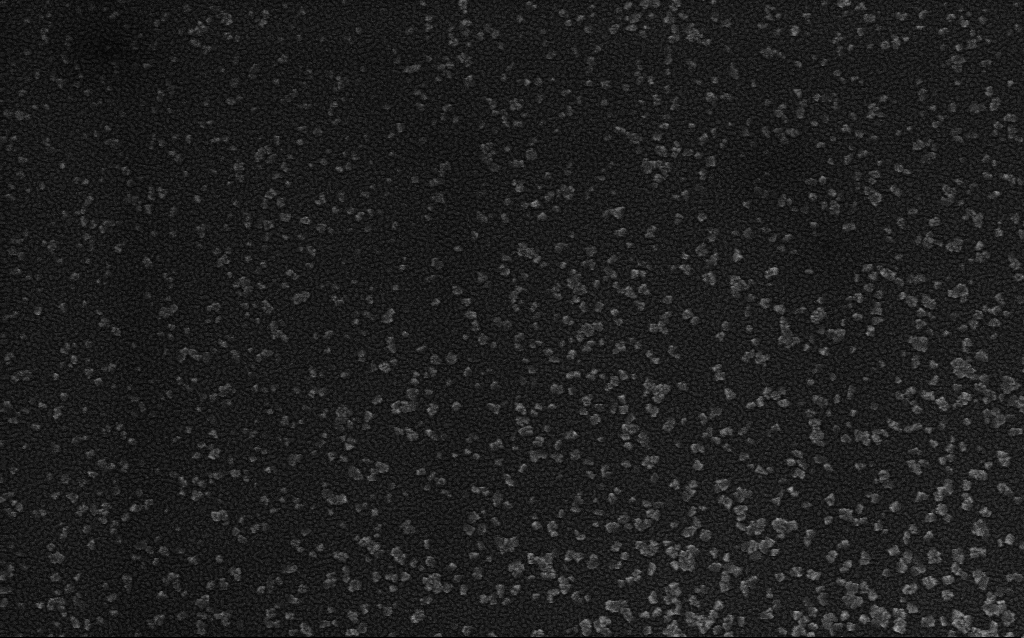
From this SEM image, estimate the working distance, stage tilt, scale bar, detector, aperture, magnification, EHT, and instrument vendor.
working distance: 1.6 mm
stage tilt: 0°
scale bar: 200 nm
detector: InLens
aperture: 30 µm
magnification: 100 K X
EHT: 4 kV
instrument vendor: Zeiss